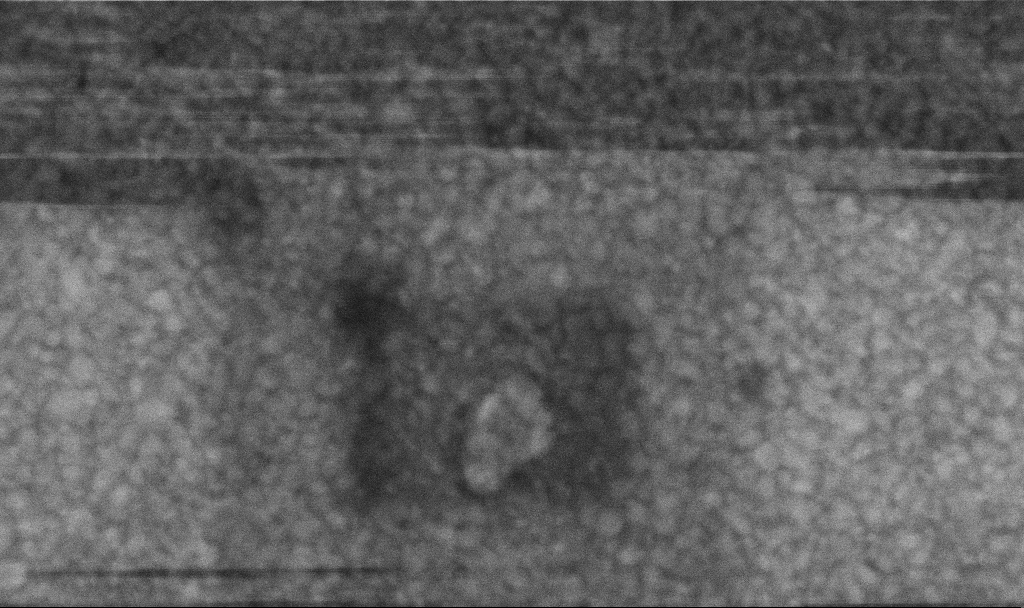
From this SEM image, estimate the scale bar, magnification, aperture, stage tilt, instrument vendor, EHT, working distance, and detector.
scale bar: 100 nm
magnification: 393.42 K X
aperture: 30 µm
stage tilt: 0°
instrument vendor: Zeiss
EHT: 5 kV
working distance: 3.2 mm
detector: InLens